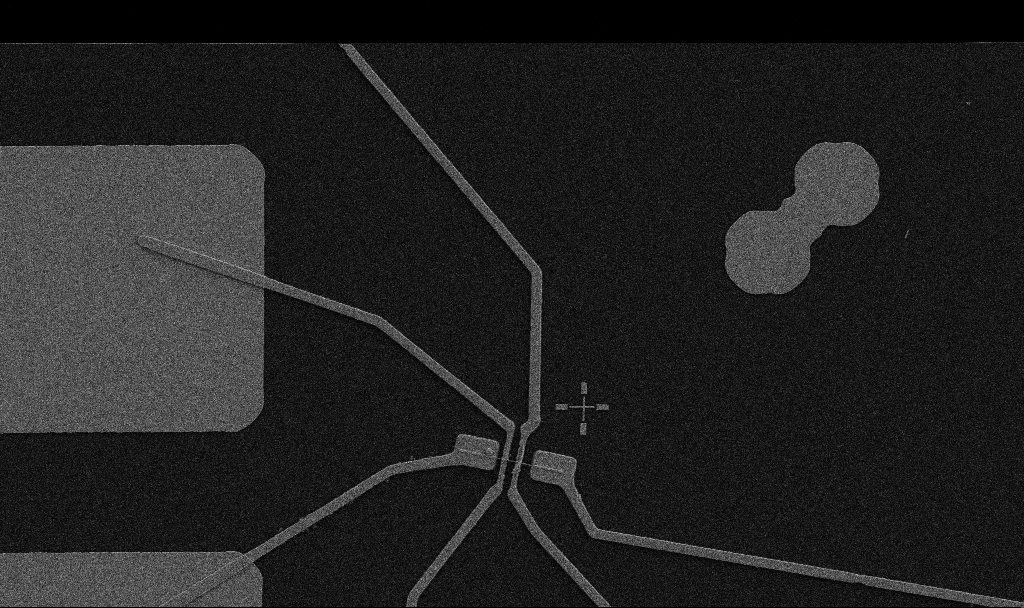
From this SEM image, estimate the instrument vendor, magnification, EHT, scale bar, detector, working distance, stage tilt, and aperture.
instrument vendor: Zeiss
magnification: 5 K X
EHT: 5 kV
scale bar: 10000 nm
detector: SE2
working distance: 10.7 mm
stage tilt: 0°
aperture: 30 µm